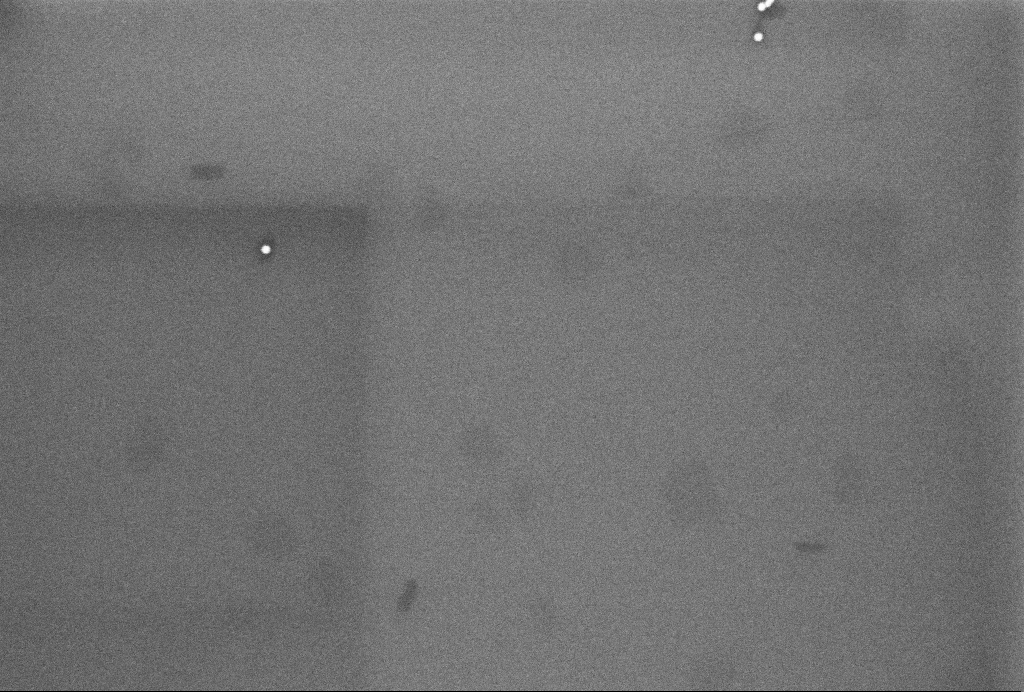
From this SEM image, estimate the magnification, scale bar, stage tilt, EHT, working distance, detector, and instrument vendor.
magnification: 108.63 K X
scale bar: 100 nm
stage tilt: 0°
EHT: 3 kV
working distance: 3.2 mm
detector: InLens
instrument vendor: Zeiss